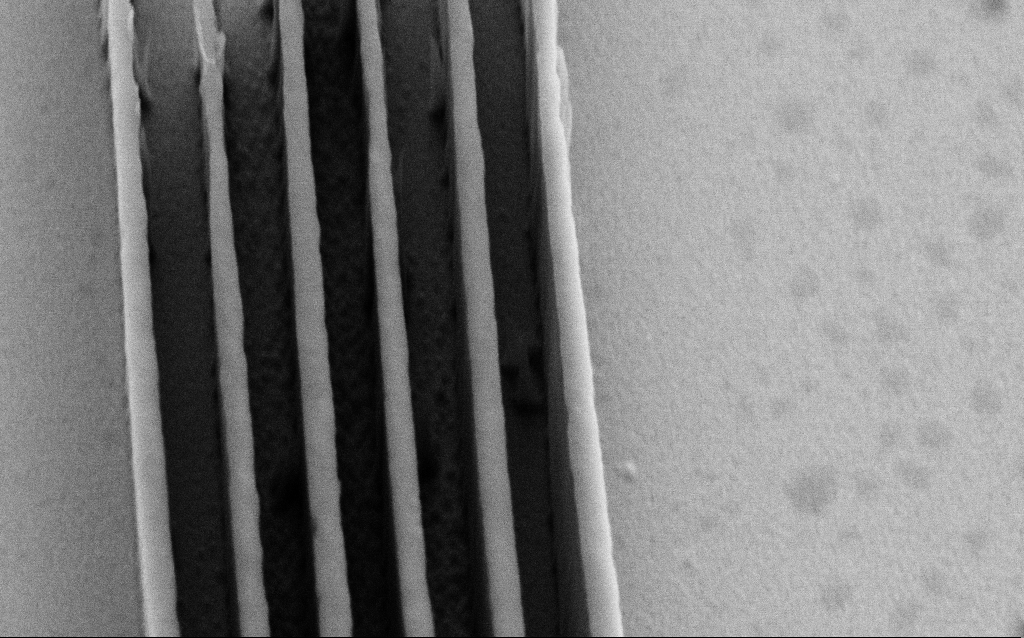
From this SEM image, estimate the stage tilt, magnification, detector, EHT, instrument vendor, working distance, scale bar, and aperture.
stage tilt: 45°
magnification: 74.21 K X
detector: SE2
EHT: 3 kV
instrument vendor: Zeiss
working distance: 9 mm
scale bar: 200 nm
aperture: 30 µm